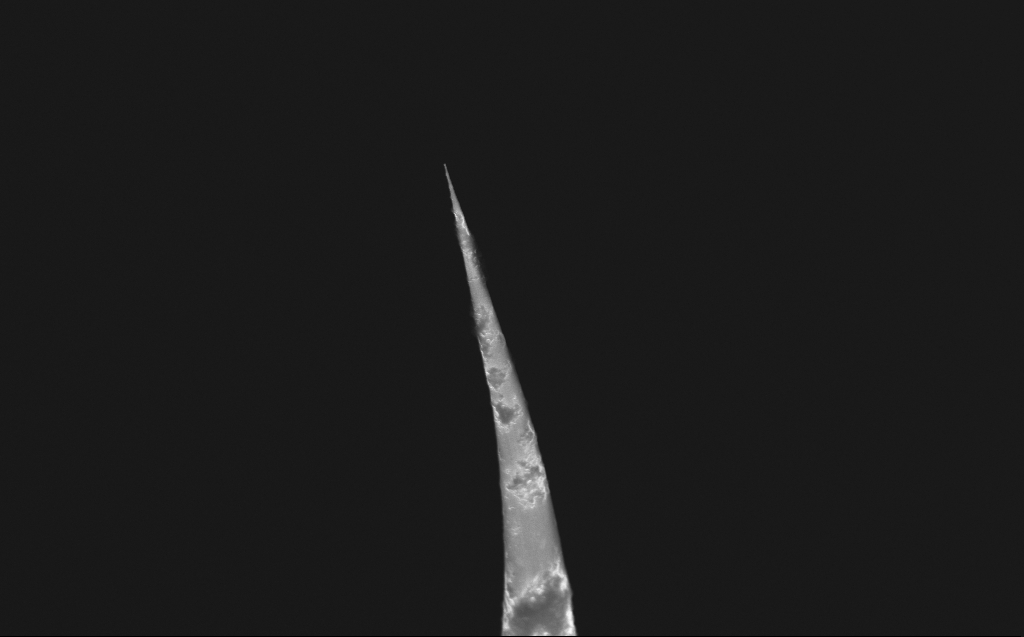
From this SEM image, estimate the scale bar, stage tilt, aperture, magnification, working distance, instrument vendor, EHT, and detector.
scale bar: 2000 nm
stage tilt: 40°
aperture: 30 µm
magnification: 20.4 K X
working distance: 6 mm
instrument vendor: Zeiss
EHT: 10 kV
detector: InLens